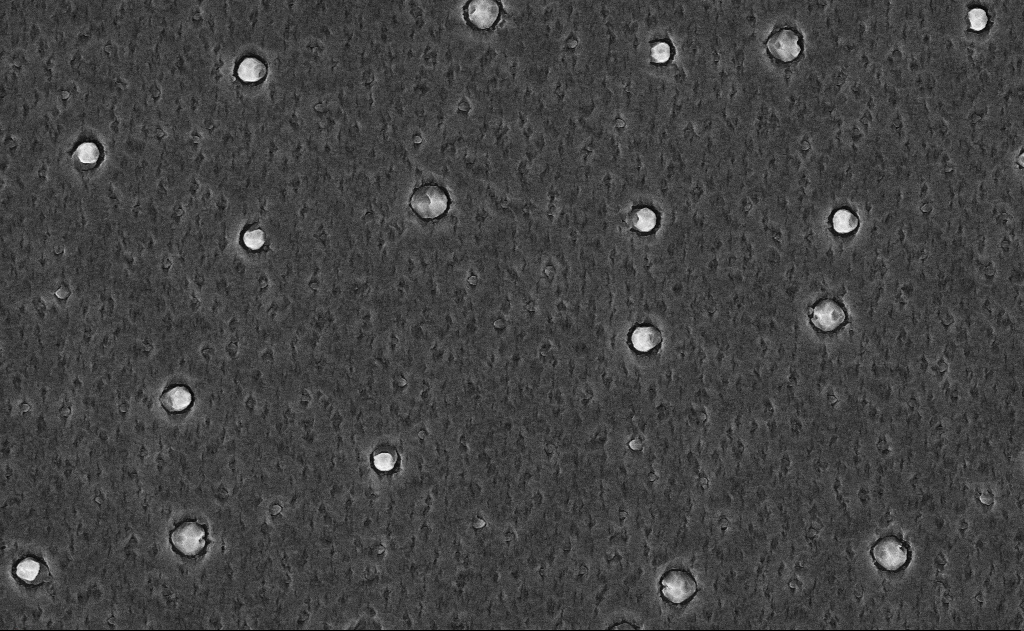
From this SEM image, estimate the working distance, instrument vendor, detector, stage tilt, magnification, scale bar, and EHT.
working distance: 18 mm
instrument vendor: Zeiss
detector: InLens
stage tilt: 0°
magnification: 20 K X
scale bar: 2000 nm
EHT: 10 kV